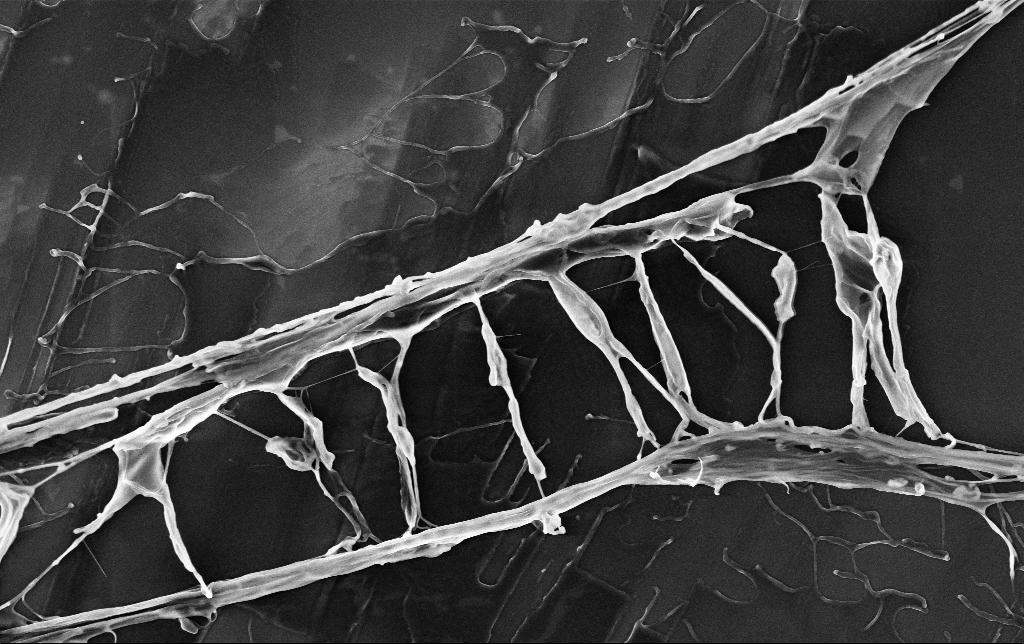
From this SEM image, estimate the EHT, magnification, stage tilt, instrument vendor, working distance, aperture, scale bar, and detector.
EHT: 3 kV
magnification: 13.84 K X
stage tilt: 0°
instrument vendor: Zeiss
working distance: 3.5 mm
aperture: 30 µm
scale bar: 2000 nm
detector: InLens